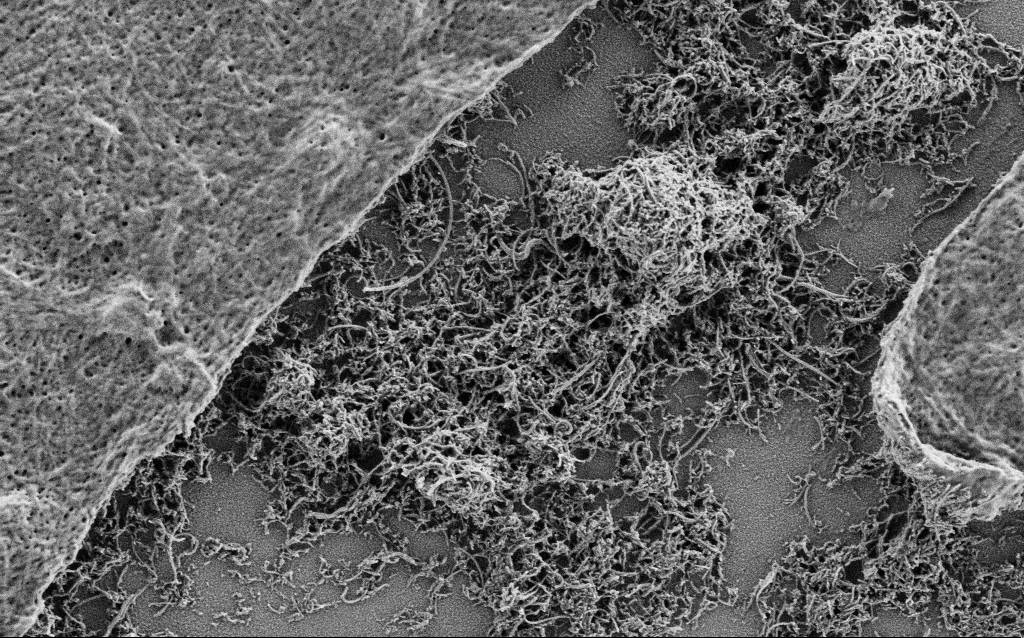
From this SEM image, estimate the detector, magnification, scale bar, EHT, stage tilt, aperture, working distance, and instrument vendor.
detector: SE2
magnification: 25 K X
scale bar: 1000 nm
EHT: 1 kV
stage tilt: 0°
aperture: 30 µm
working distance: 4 mm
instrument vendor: Zeiss